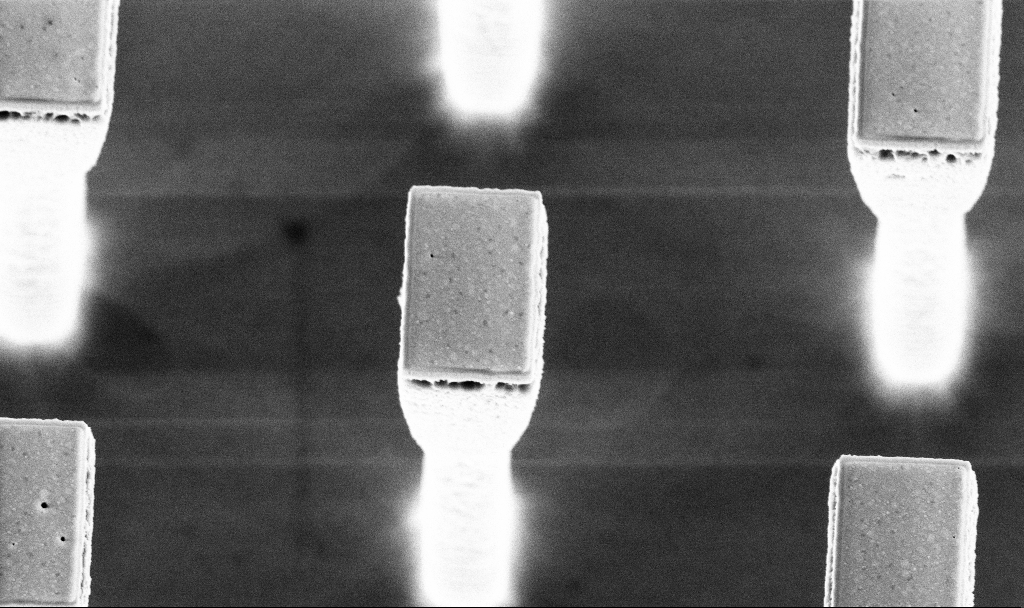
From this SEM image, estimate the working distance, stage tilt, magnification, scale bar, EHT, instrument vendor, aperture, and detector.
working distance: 4.2 mm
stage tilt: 20.2°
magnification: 16.21 K X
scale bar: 1000 nm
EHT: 5 kV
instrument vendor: Zeiss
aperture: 30 µm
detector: InLens